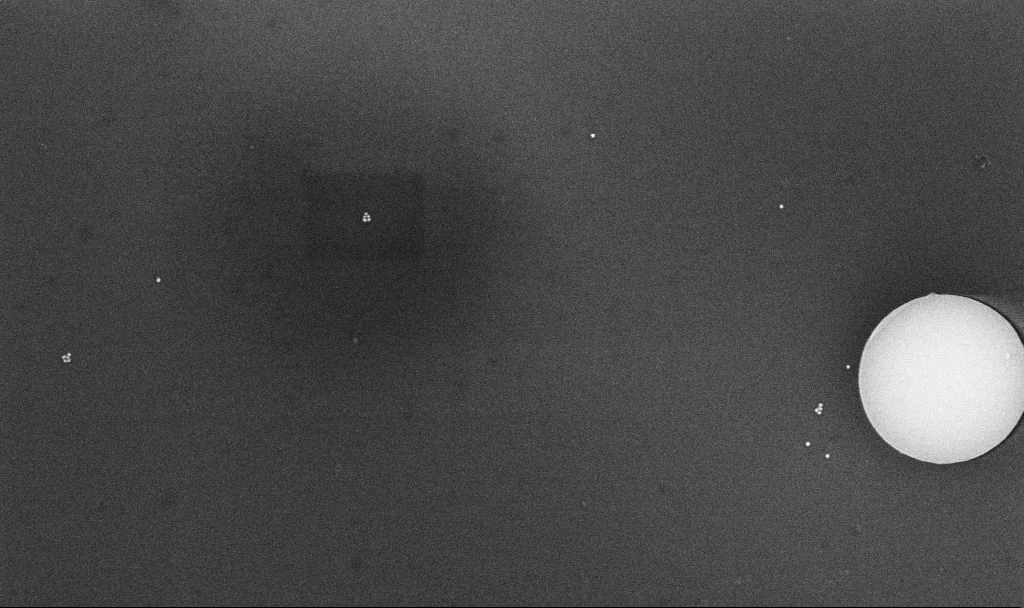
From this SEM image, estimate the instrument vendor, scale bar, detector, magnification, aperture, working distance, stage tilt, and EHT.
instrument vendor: Zeiss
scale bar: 200 nm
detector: InLens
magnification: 75.71 K X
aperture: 30 µm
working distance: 4.3 mm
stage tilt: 0°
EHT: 10 kV